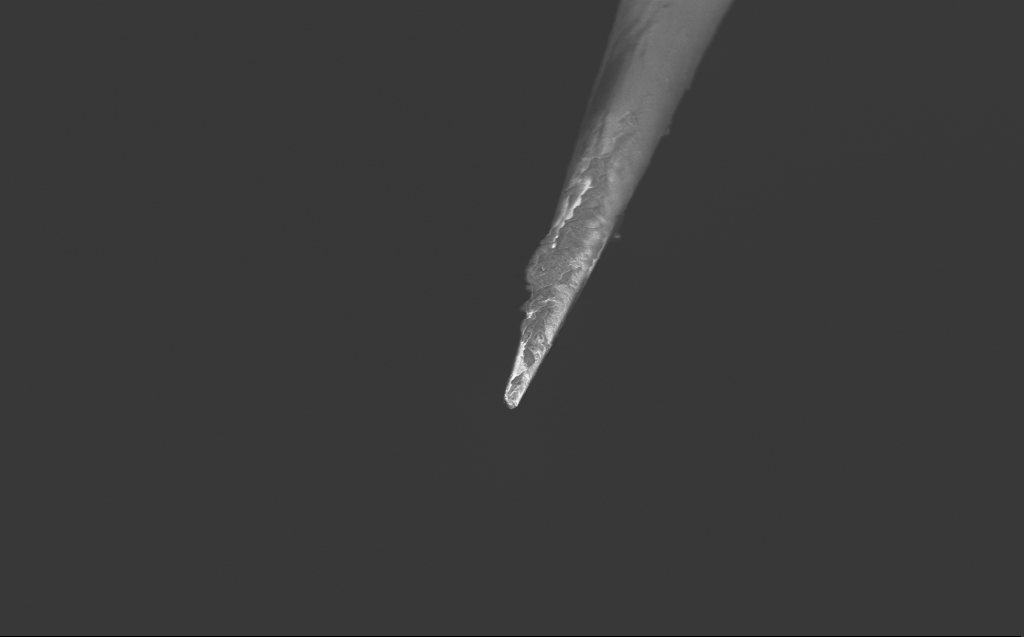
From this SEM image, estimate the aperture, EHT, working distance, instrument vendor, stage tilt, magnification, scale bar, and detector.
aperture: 30 µm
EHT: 2 kV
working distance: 3 mm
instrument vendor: Zeiss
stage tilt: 45°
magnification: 10 K X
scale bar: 2000 nm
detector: InLens